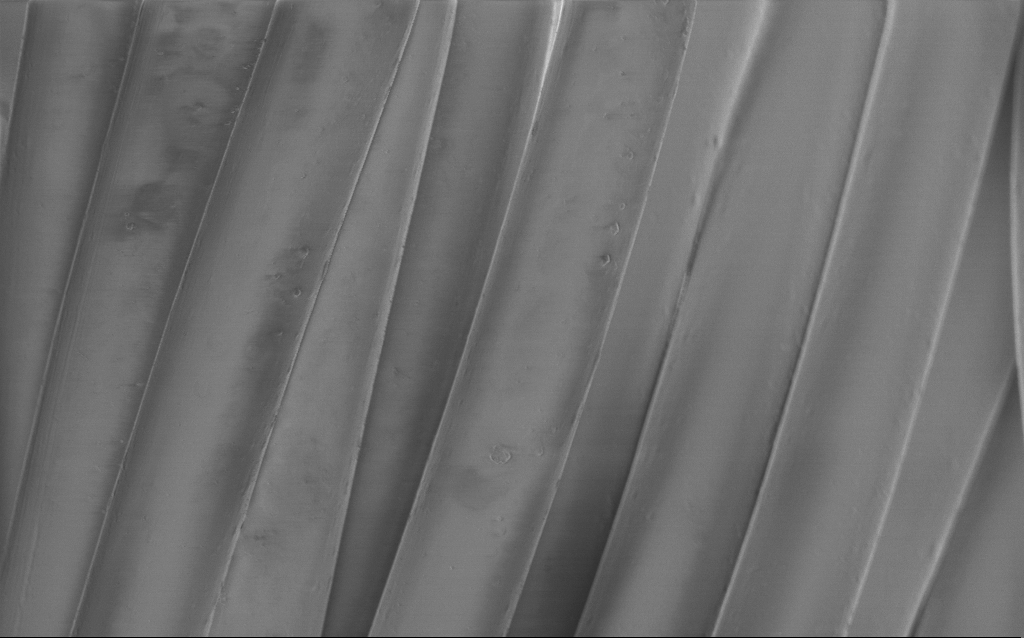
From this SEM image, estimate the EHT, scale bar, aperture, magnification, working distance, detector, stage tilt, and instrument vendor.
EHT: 1 kV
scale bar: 10000 nm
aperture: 30 µm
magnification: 2.07 K X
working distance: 4 mm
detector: InLens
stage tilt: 0°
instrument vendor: Zeiss